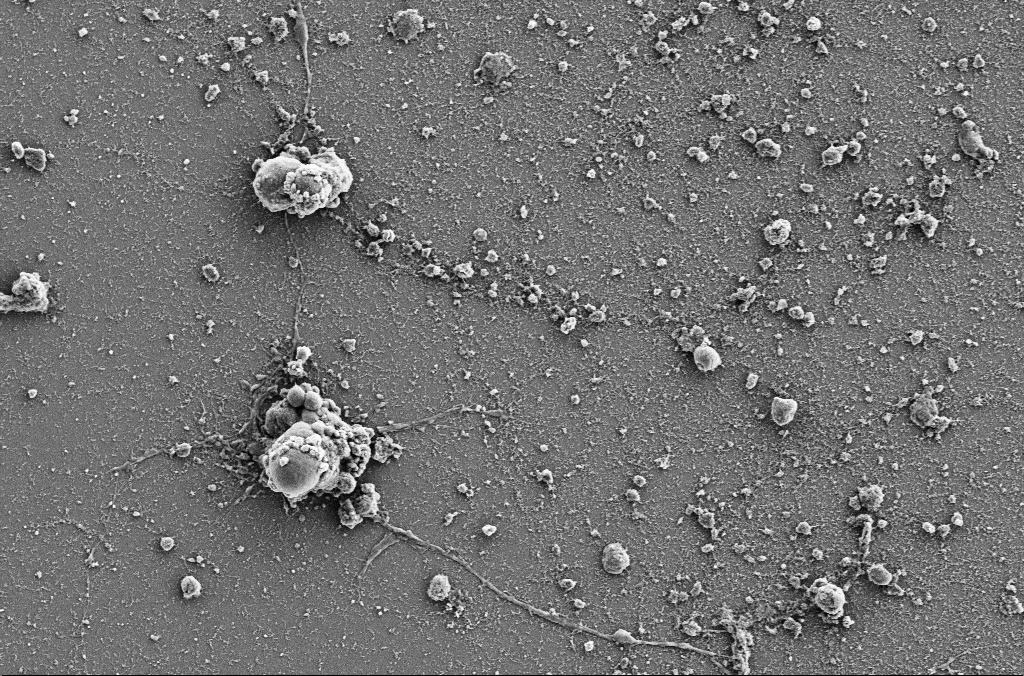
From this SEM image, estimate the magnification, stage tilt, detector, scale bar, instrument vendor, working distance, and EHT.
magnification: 2.5 K X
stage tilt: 0°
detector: SE2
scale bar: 10000 nm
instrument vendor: Zeiss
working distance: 4 mm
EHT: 5 kV